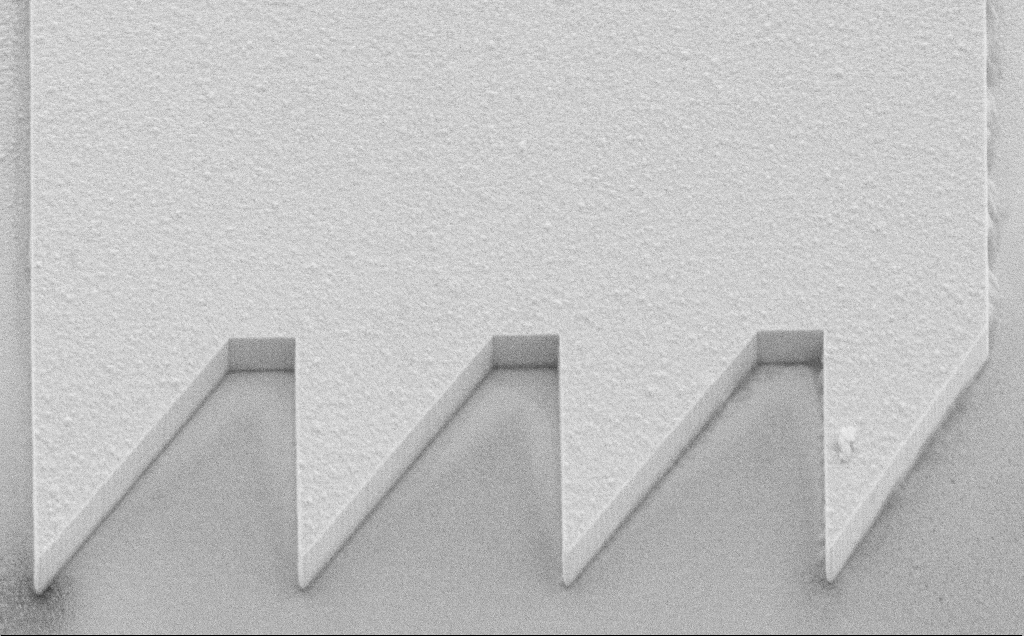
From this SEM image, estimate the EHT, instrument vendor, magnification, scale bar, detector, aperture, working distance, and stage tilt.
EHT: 10 kV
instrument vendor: Zeiss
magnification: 8.4 K X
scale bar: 2000 nm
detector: SE2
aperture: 30 µm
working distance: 8 mm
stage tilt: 45°